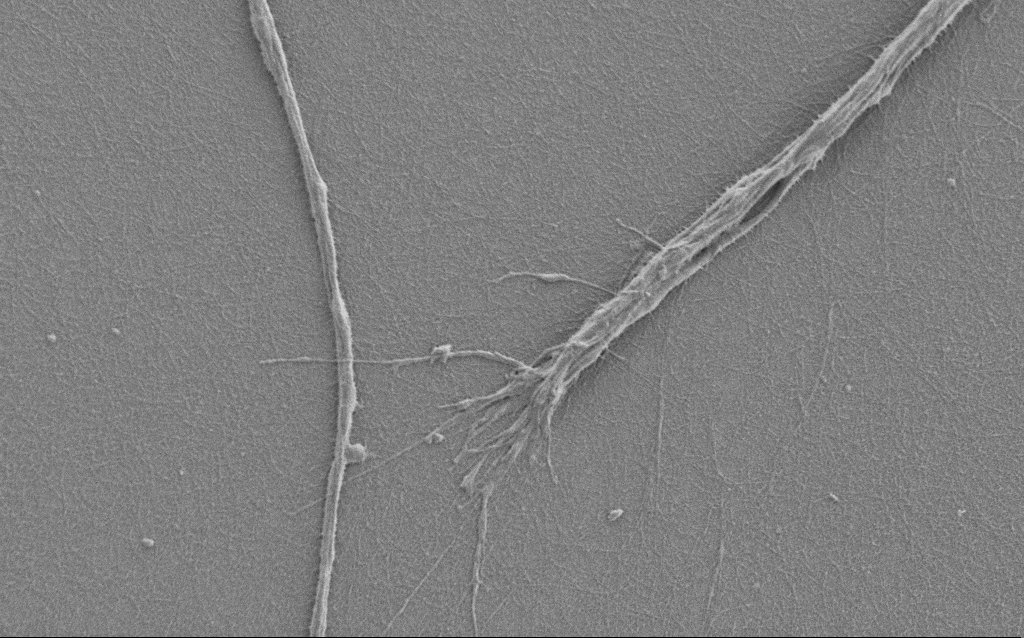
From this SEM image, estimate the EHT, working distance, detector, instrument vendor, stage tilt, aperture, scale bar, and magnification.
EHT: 1 kV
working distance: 6 mm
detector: SE2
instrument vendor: Zeiss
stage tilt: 0°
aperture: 30 µm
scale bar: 2000 nm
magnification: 7.5 K X